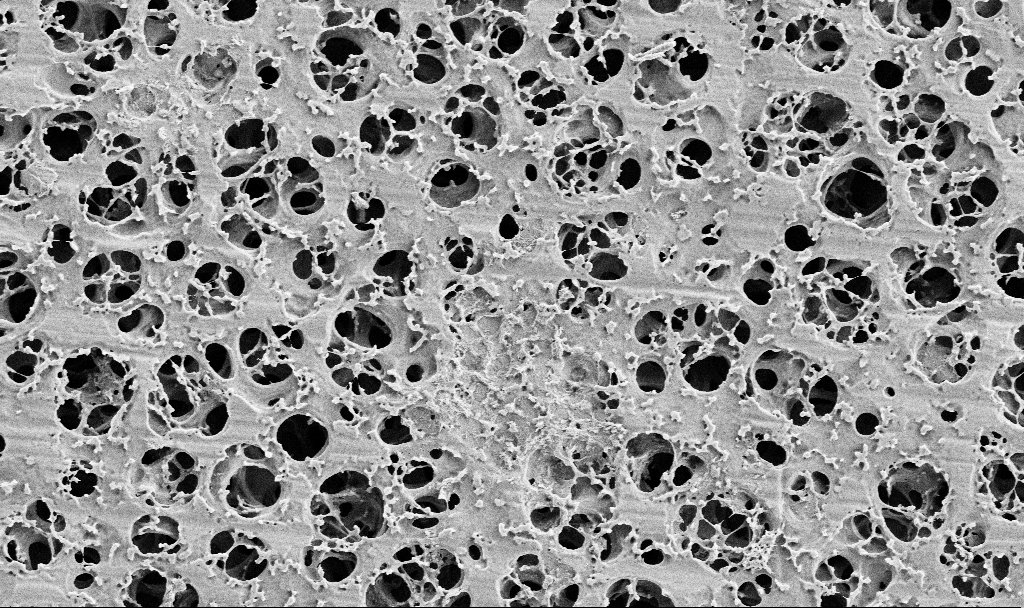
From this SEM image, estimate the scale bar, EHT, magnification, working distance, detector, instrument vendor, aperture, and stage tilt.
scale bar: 2000 nm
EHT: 2 kV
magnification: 10 K X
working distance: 3.6 mm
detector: SE2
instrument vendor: Zeiss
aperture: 30 µm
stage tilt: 0°